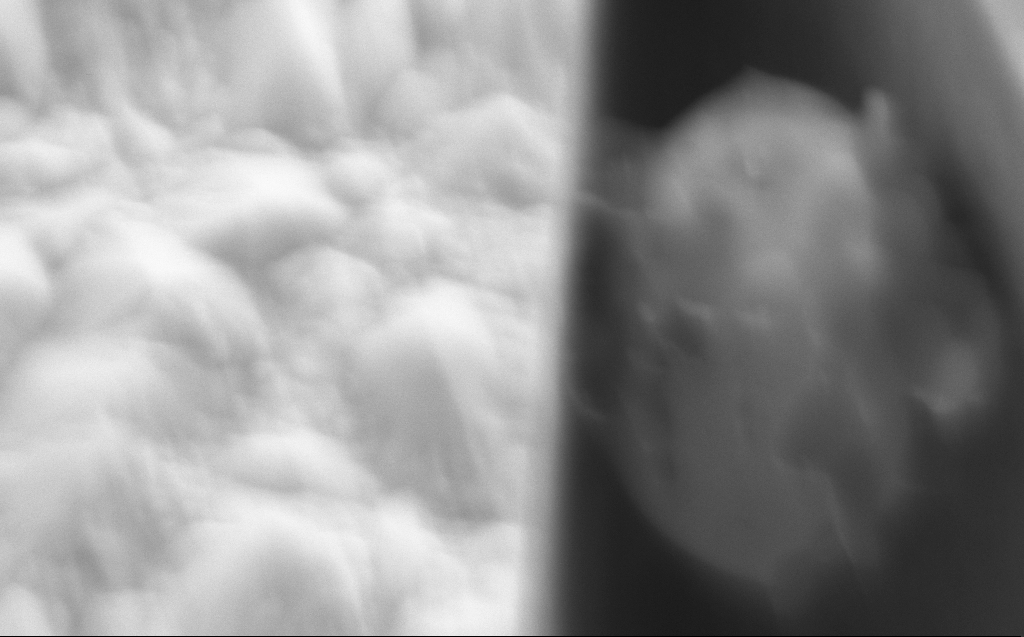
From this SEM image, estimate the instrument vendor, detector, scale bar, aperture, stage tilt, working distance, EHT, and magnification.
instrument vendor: Zeiss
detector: SE2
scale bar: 200 nm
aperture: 120 µm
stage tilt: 45.1°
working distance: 7 mm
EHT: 10 kV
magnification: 72.69 K X